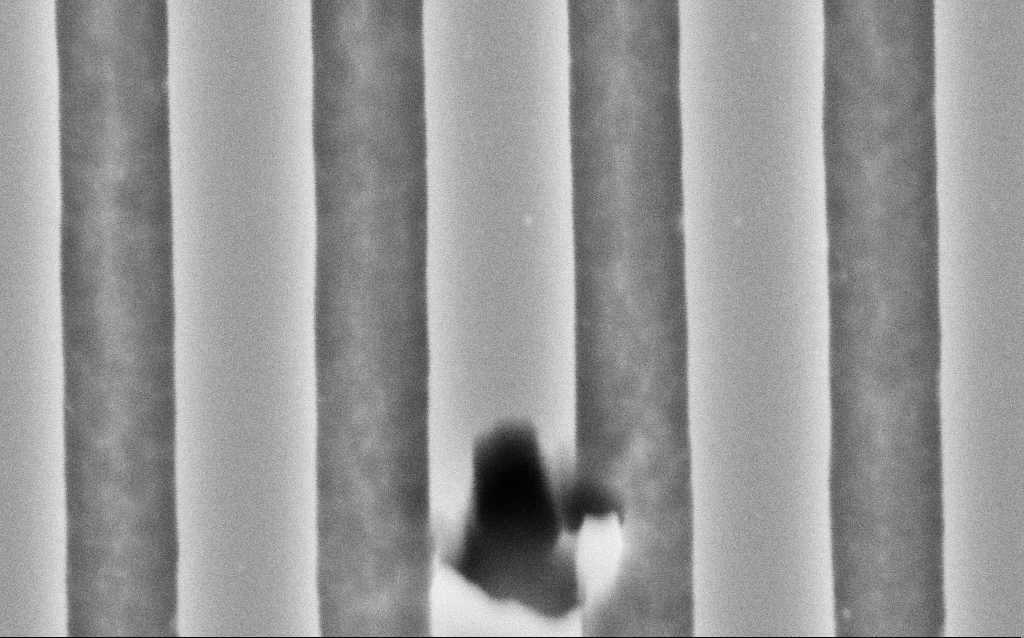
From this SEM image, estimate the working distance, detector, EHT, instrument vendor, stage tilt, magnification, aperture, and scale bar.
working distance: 6 mm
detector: SE2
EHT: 3 kV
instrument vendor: Zeiss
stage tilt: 45°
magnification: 189.83 K X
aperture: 30 µm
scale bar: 200 nm